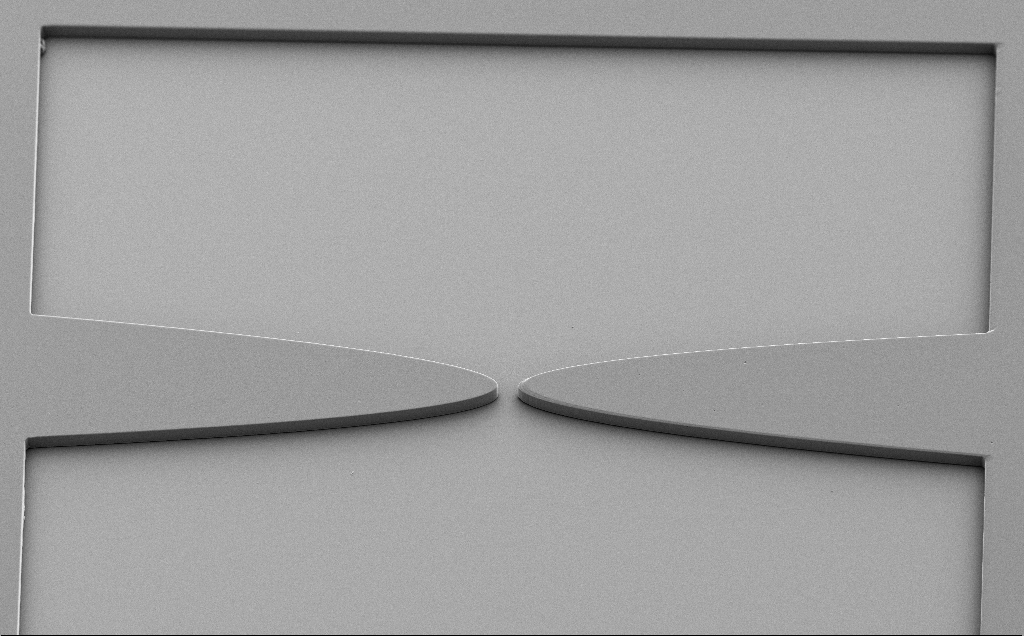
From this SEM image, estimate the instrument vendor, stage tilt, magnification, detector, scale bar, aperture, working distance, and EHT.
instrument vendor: Zeiss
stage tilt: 40°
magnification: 0.64 K X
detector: SE2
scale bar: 100000 nm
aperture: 30 µm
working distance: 9 mm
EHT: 5 kV